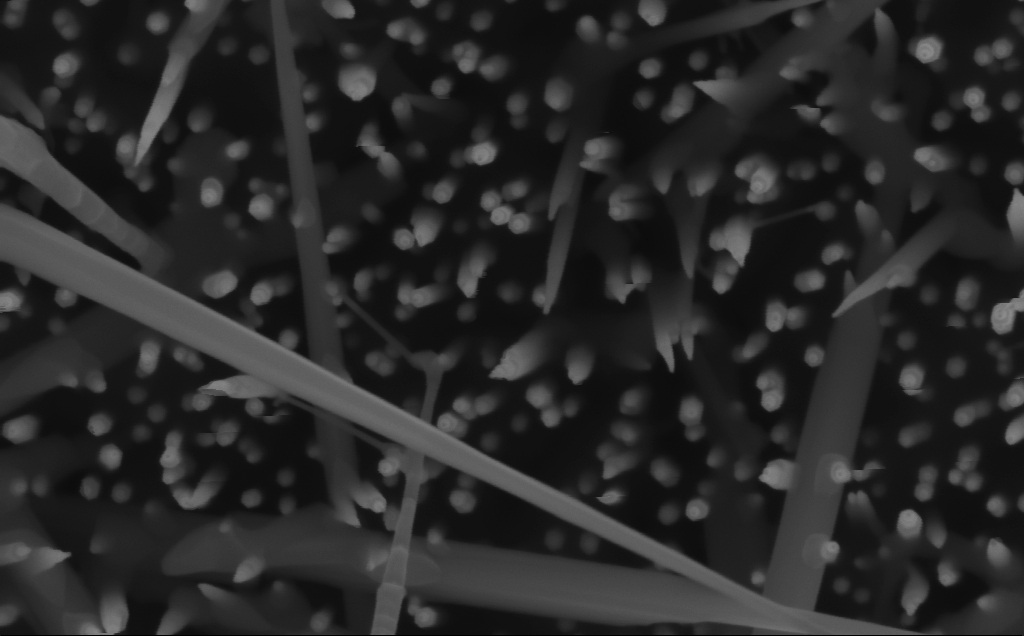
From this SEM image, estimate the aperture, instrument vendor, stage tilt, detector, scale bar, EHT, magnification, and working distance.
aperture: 30 µm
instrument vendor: Zeiss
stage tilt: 0°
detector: InLens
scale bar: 100 nm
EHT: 10 kV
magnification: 188.31 K X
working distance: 6 mm